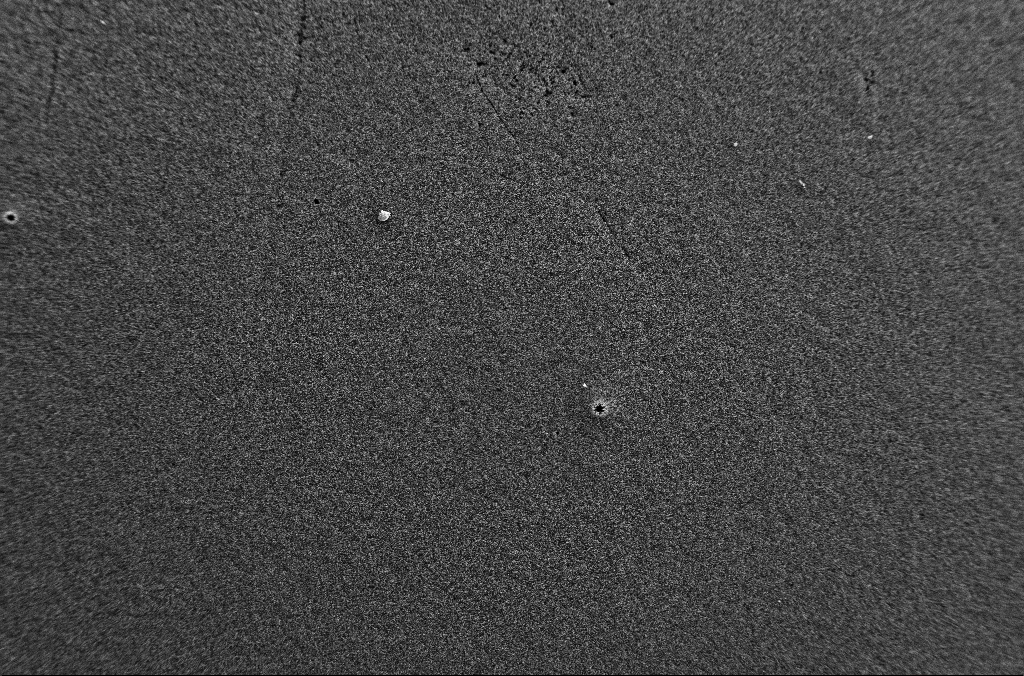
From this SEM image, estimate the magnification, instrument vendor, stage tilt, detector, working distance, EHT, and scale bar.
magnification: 0.25 K X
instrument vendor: Zeiss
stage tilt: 0°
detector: SE2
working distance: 5.3 mm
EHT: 1.8 kV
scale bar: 100000 nm